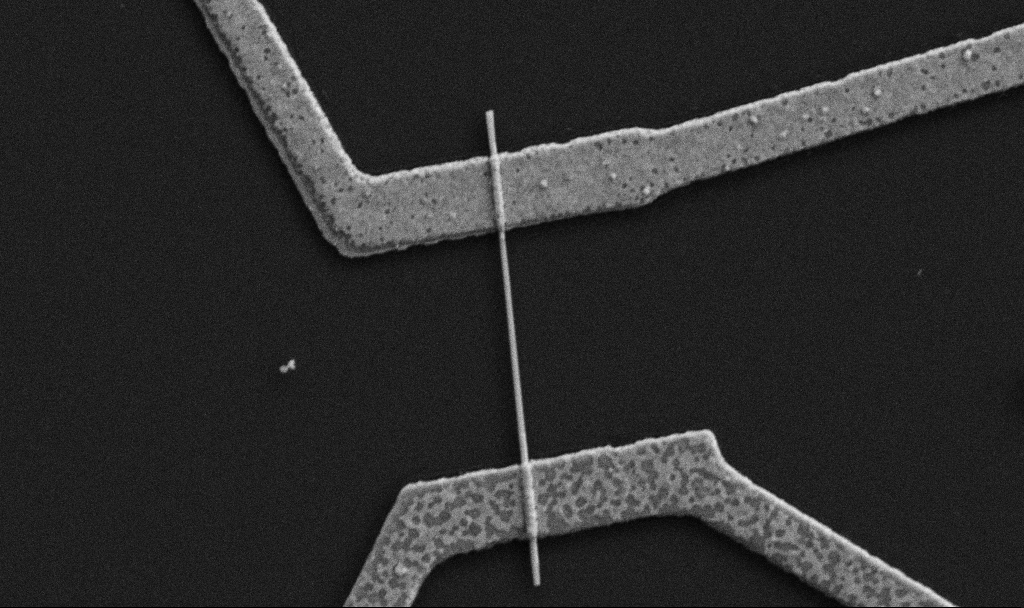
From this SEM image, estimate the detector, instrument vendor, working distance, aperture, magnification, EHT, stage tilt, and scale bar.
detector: SE2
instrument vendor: Zeiss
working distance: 8.7 mm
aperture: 30 µm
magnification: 30 K X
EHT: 5 kV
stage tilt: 0°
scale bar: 1000 nm